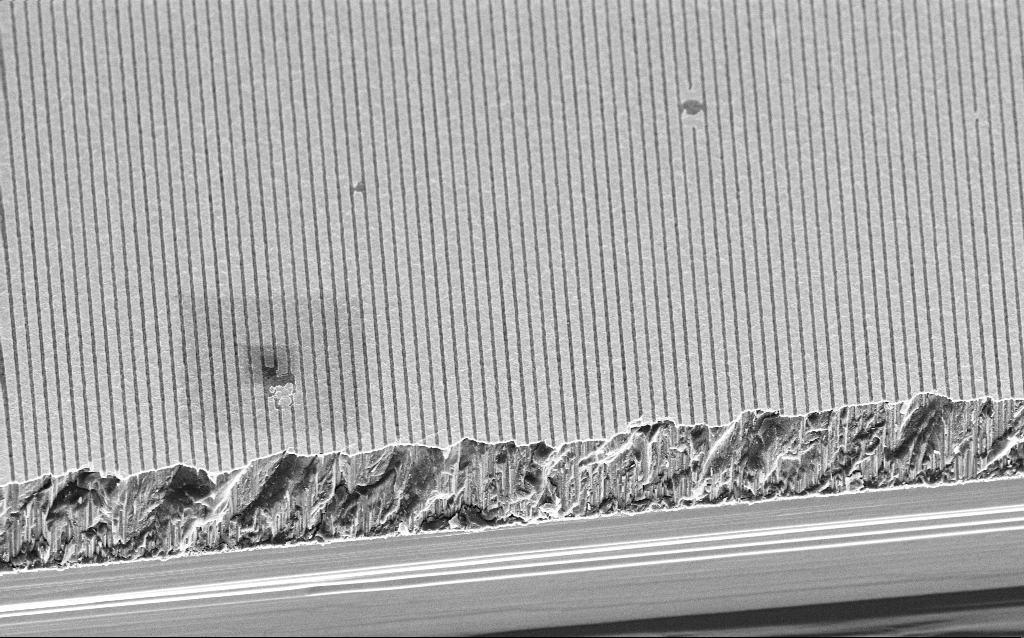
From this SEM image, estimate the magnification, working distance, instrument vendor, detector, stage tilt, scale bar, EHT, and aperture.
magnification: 9.54 K X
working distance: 6 mm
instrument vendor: Zeiss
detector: InLens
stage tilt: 45°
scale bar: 2000 nm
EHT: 3 kV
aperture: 30 µm